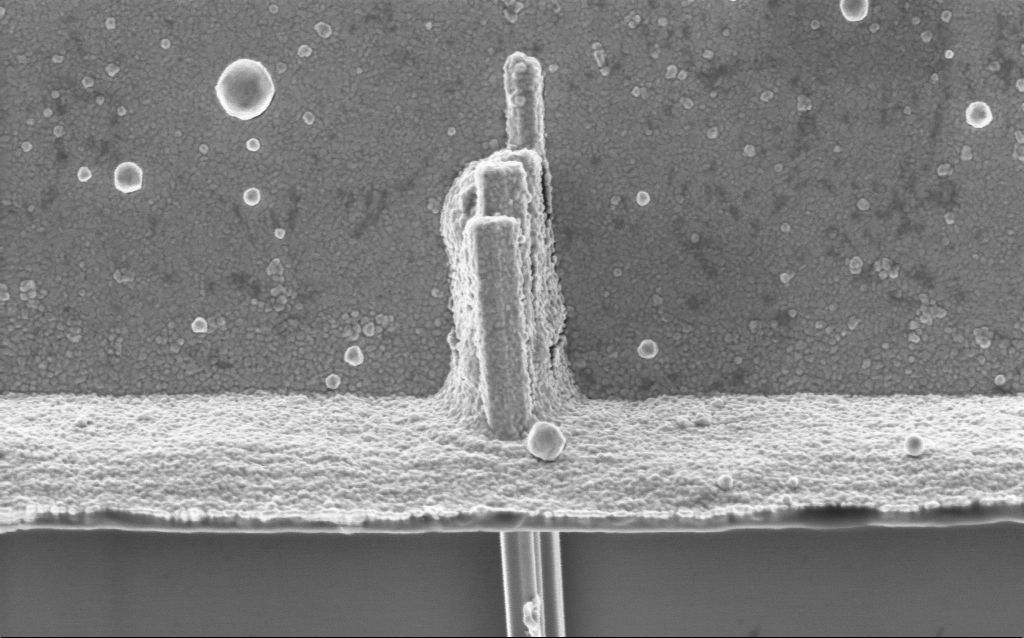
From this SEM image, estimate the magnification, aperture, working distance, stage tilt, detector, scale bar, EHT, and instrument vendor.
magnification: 63.69 K X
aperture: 30 µm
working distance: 7.7 mm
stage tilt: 0°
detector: InLens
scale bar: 1000 nm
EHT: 2 kV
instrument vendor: Zeiss